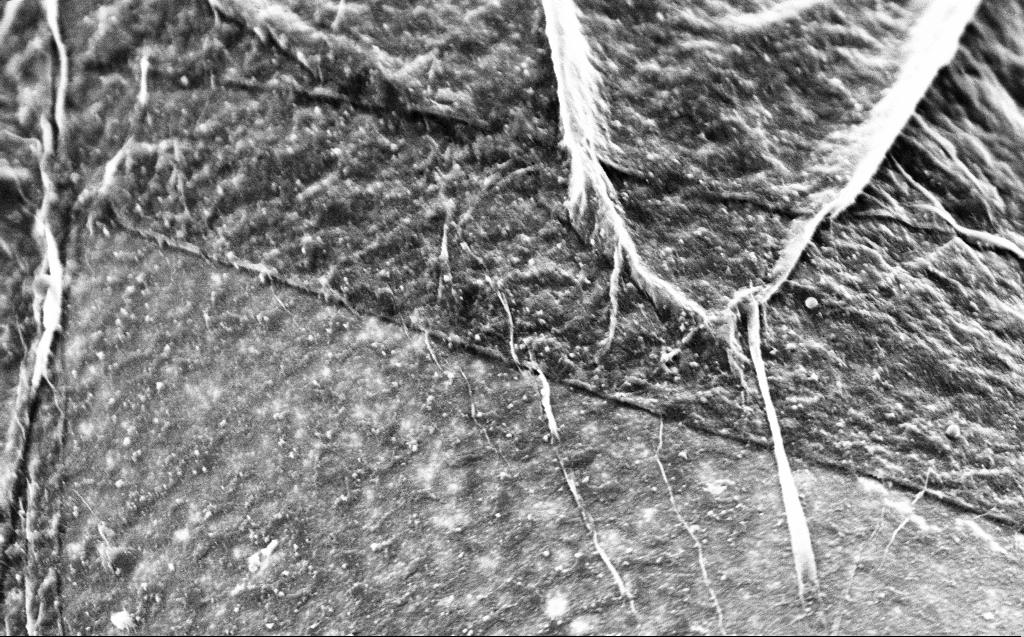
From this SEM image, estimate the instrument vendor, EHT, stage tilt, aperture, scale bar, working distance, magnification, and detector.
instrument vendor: Zeiss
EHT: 2 kV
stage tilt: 45°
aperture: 30 µm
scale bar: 1000 nm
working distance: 3 mm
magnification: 50 K X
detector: InLens